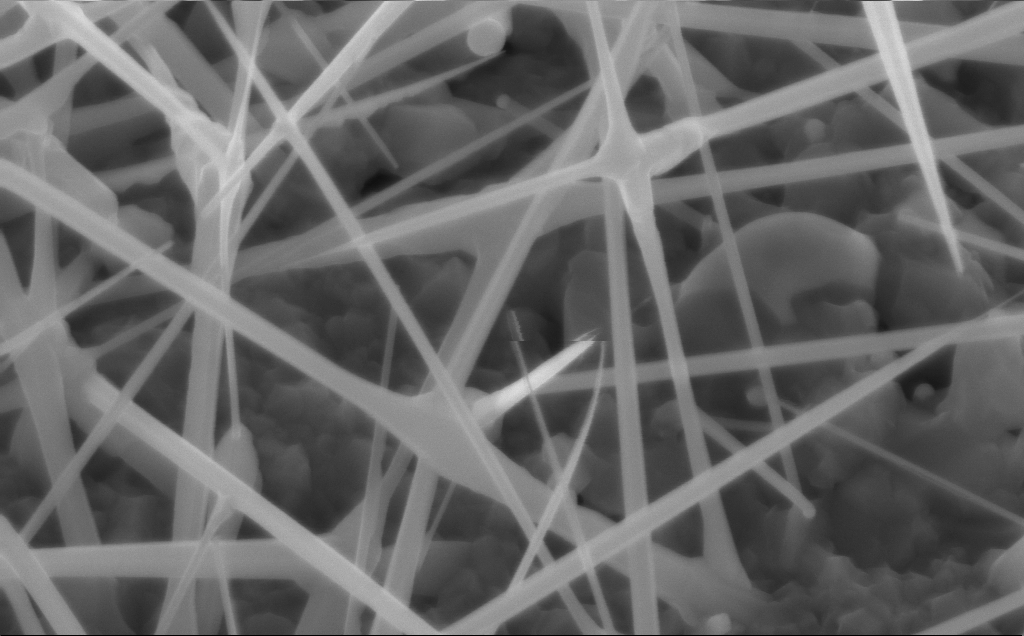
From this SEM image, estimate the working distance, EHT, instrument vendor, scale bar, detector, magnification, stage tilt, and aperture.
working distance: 5 mm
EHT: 10 kV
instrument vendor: Zeiss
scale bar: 200 nm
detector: InLens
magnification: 80 K X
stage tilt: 30°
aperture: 30 µm